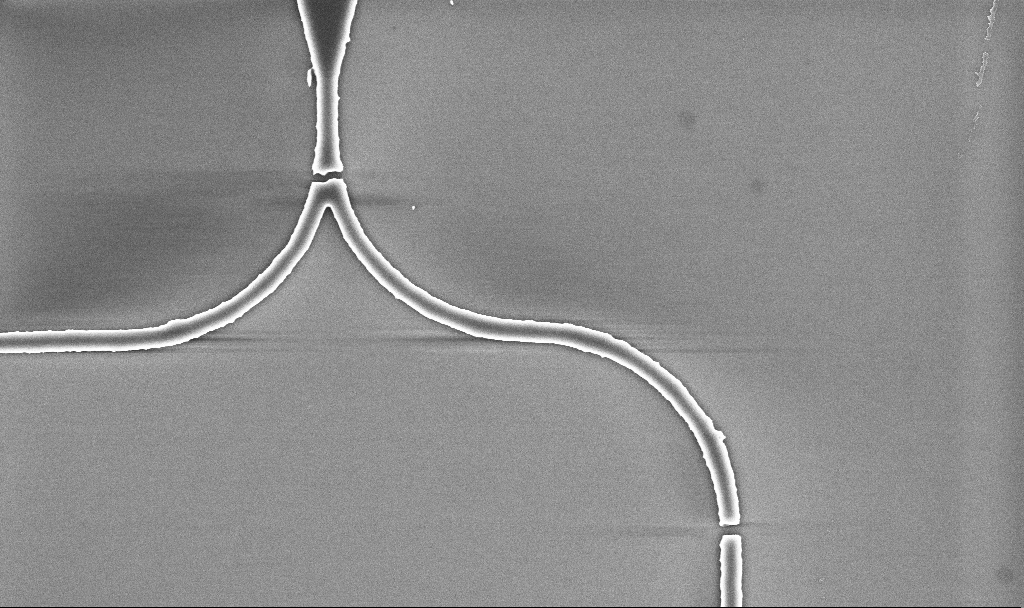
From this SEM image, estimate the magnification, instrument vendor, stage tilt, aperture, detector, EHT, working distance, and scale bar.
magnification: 15.66 K X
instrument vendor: Zeiss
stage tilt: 0°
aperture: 30 µm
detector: InLens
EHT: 5 kV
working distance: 5.2 mm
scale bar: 2000 nm